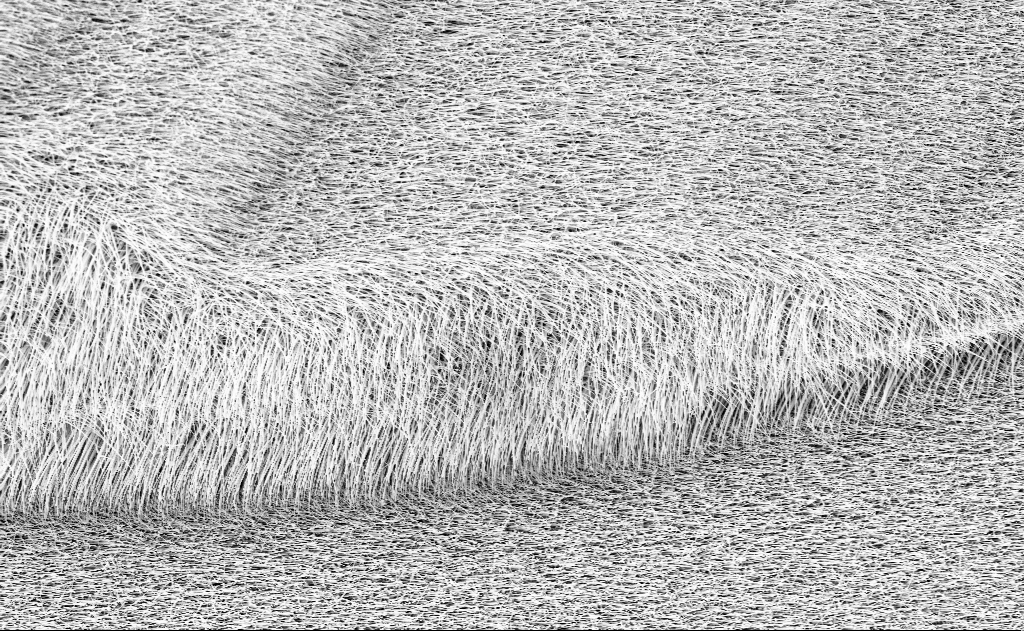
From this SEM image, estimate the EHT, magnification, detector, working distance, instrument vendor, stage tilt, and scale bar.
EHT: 10 kV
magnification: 5 K X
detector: InLens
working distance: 13 mm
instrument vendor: Zeiss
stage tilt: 0°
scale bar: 10000 nm